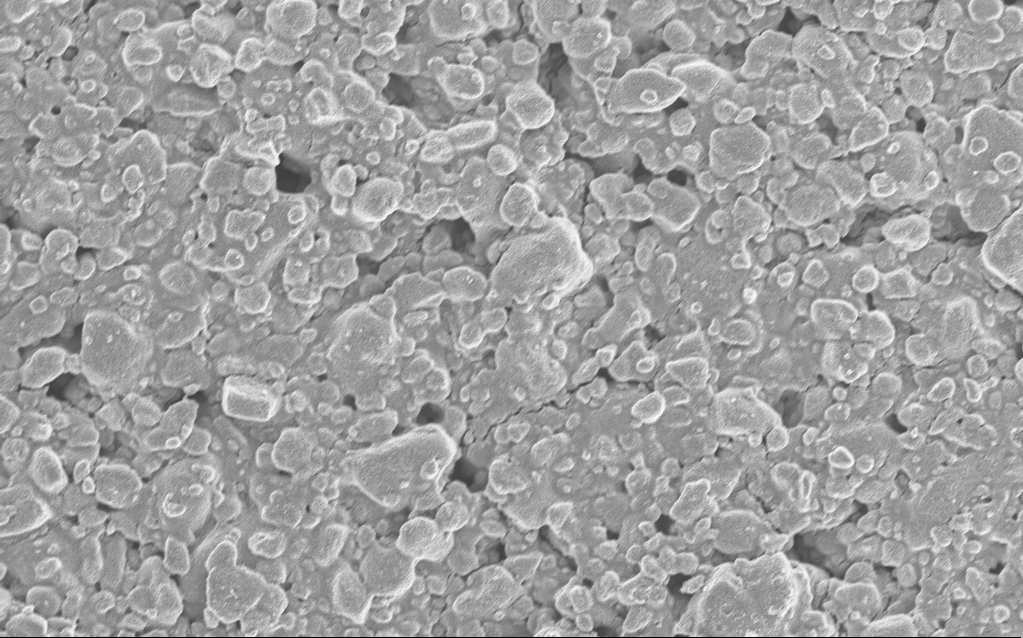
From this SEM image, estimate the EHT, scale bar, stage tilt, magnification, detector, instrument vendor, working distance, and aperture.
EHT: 5 kV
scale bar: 10000 nm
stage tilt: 0°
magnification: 3.88 K X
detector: InLens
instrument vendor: Zeiss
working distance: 4.8 mm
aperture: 30 µm